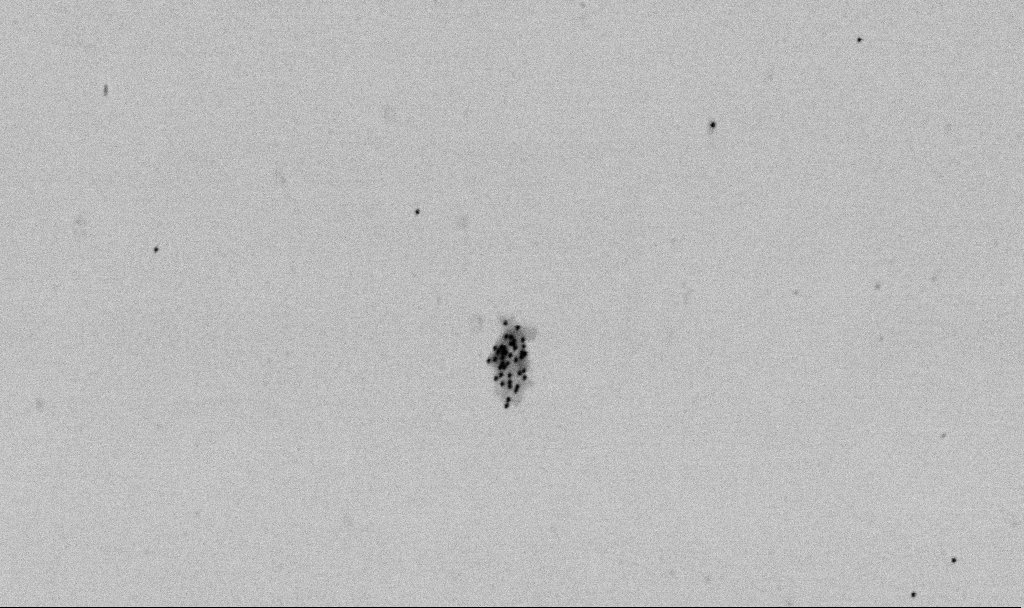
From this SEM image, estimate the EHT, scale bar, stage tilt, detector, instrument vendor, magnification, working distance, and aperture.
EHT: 2 kV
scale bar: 1000 nm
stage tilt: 0°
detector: SE2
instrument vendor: Zeiss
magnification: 50 K X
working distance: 6.5 mm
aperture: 30 µm